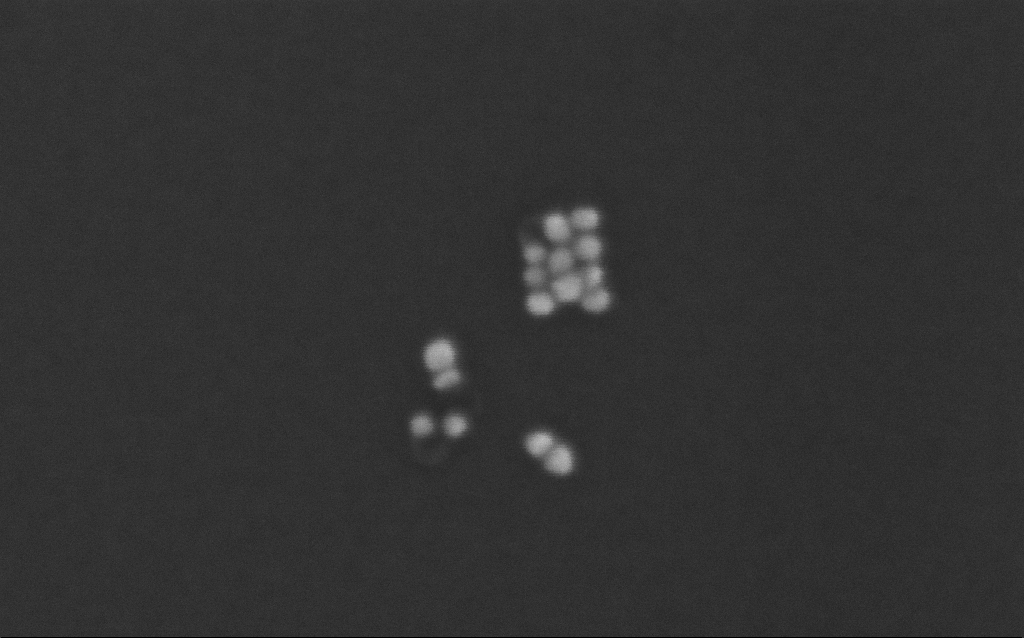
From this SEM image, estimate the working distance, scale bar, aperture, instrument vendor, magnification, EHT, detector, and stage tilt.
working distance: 7 mm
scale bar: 100 nm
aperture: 30 µm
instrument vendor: Zeiss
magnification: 643.08 K X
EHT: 10 kV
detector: InLens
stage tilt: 0°